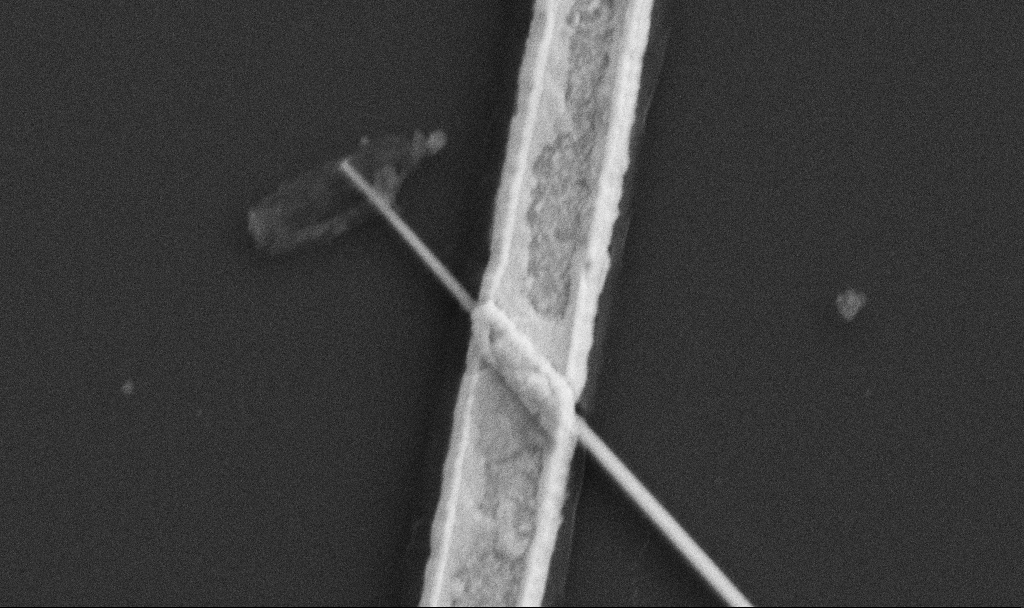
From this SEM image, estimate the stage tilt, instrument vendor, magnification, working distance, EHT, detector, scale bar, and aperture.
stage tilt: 0°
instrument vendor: Zeiss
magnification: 60 K X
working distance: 10.7 mm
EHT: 5 kV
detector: SE2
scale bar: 1000 nm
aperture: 30 µm